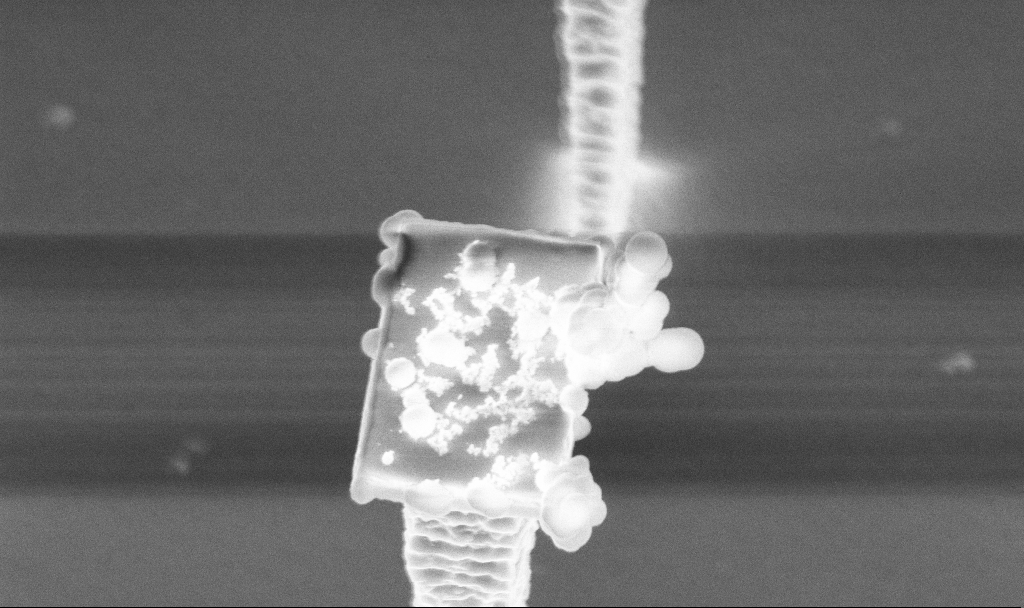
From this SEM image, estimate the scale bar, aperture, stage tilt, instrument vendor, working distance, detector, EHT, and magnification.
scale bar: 2000 nm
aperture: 30 µm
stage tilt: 30°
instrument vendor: Zeiss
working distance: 5 mm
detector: InLens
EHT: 5 kV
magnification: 26.35 K X